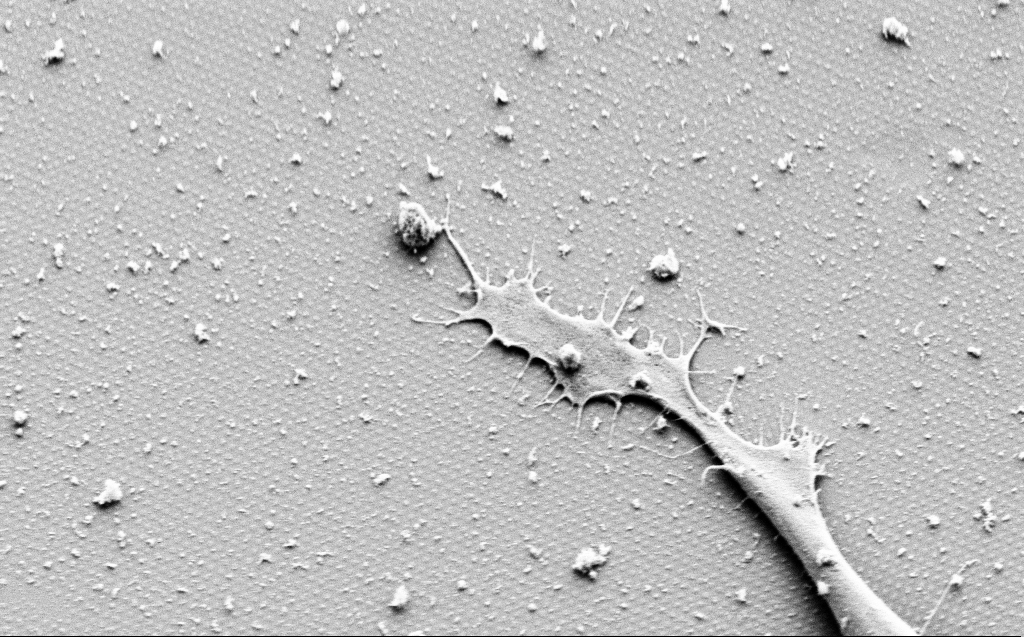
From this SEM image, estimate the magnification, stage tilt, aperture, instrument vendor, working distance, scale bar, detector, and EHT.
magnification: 7.92 K X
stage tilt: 45°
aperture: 30 µm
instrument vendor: Zeiss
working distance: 9 mm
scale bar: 2000 nm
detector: SE2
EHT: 3 kV